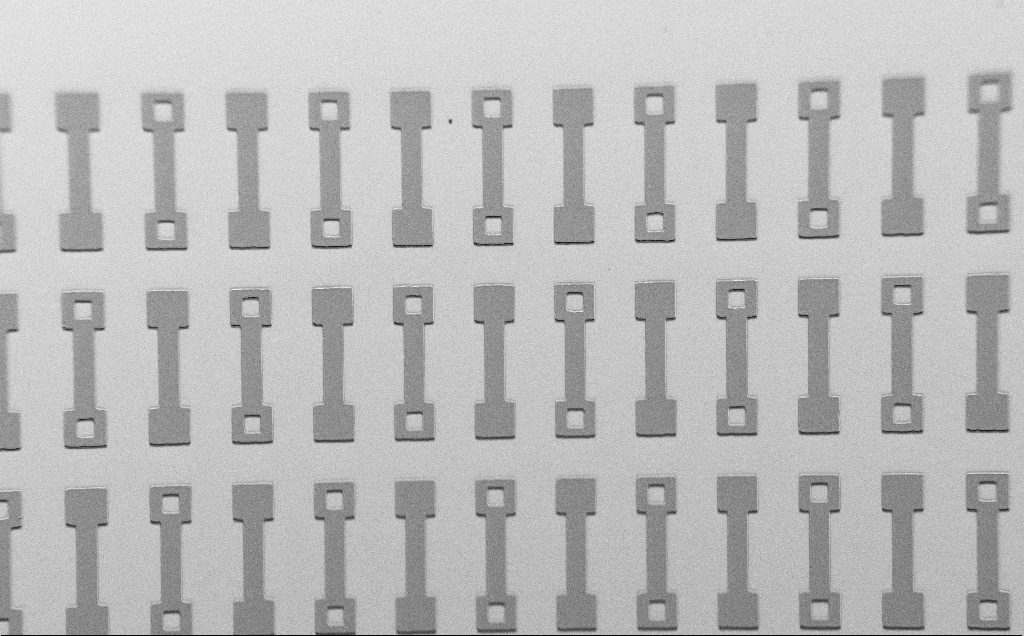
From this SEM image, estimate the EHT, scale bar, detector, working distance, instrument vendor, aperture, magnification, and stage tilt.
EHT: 1.5 kV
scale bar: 100000 nm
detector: SE2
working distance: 7 mm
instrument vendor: Zeiss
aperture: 30 µm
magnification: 0.295 K X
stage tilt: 45°